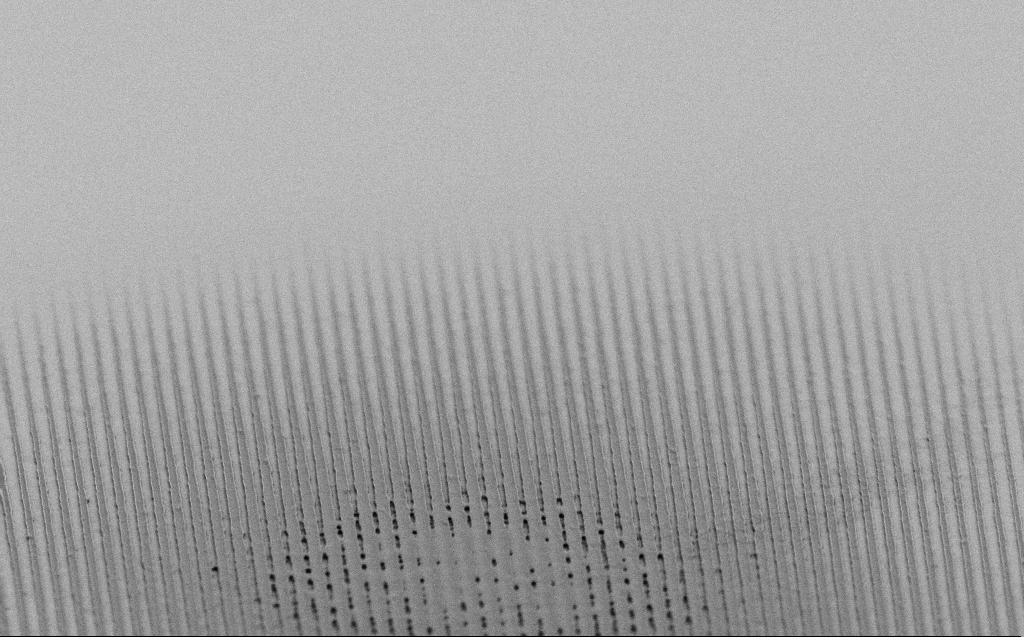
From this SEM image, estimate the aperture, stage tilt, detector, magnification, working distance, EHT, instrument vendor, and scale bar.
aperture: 30 µm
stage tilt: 60.7°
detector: SE2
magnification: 13.91 K X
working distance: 3 mm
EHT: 1 kV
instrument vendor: Zeiss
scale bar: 2000 nm